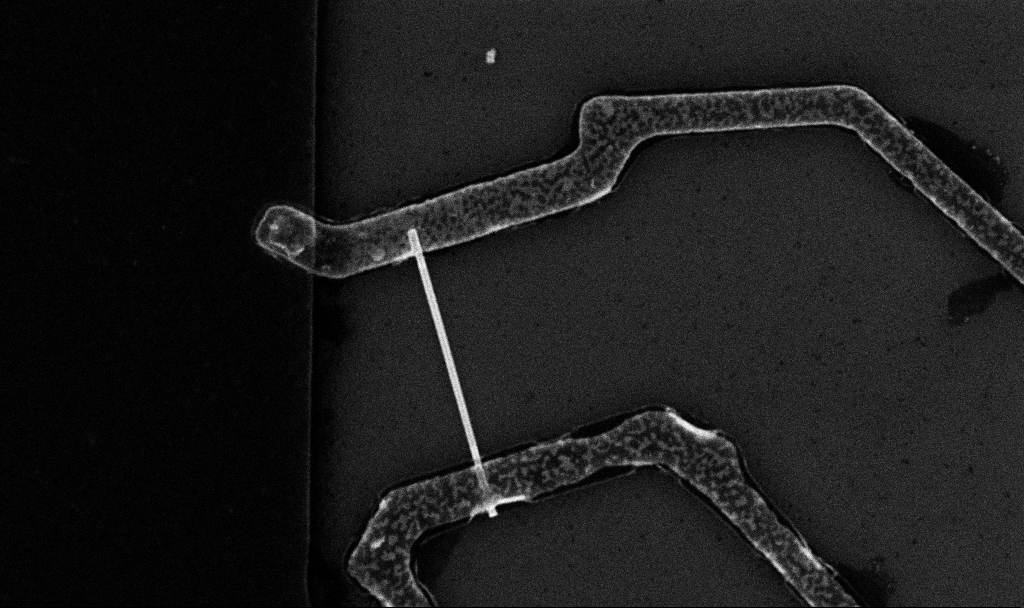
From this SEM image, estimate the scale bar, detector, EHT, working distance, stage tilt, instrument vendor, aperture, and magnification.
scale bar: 2000 nm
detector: InLens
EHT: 10 kV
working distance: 6.7 mm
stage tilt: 0°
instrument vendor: Zeiss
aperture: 30 µm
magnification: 20 K X